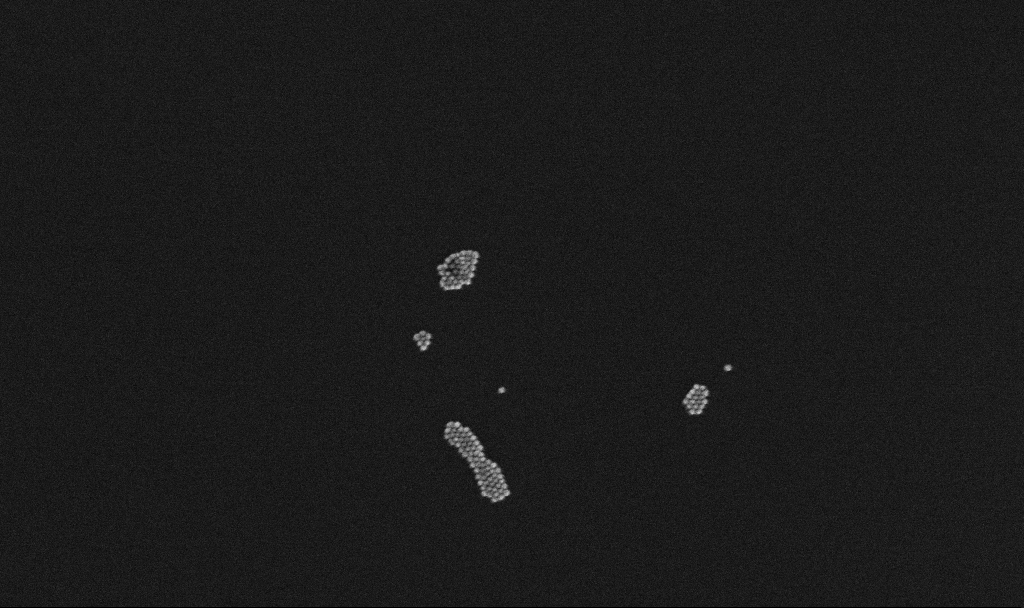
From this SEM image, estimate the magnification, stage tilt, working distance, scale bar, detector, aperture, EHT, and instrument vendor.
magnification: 100 K X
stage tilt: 0°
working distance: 3.3 mm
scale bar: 200 nm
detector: InLens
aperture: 30 µm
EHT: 10 kV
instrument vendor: Zeiss